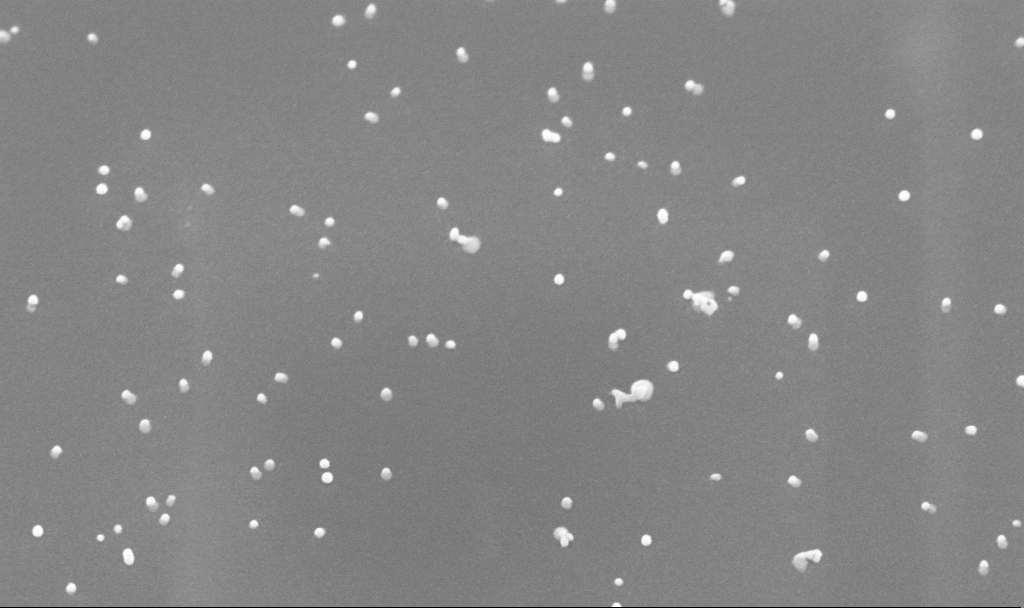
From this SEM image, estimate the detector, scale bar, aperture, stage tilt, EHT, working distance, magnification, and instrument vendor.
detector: InLens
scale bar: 200 nm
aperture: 30 µm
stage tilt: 45°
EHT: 10 kV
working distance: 3.8 mm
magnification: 150 K X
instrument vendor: Zeiss